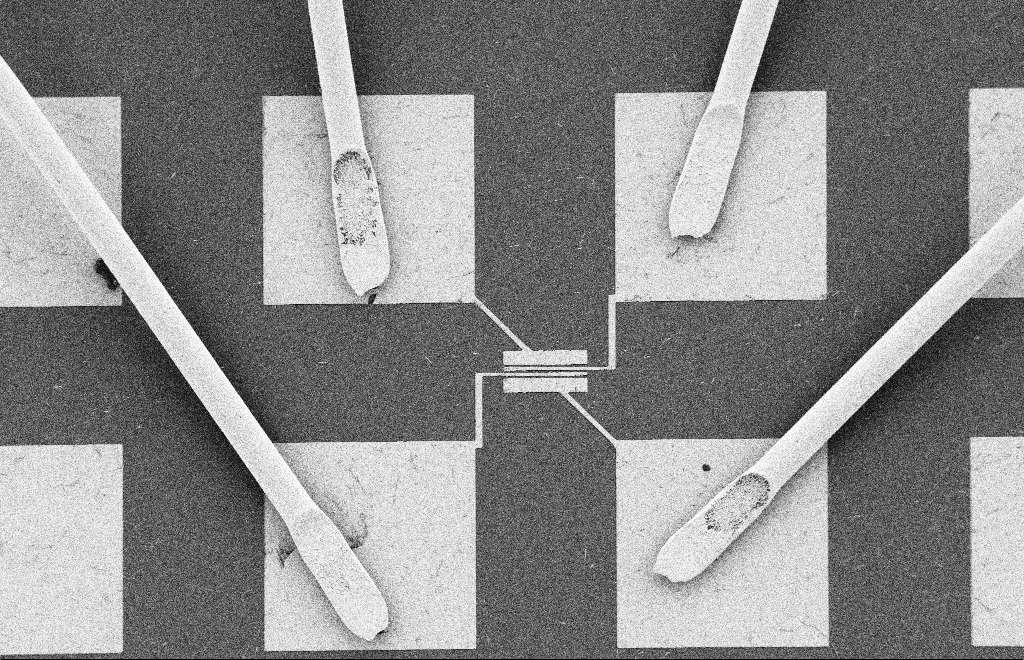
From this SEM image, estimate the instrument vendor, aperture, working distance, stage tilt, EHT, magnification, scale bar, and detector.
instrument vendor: Zeiss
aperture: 20 µm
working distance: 10 mm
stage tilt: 0°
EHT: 2 kV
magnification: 0.508 K X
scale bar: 20000 nm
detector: SE2